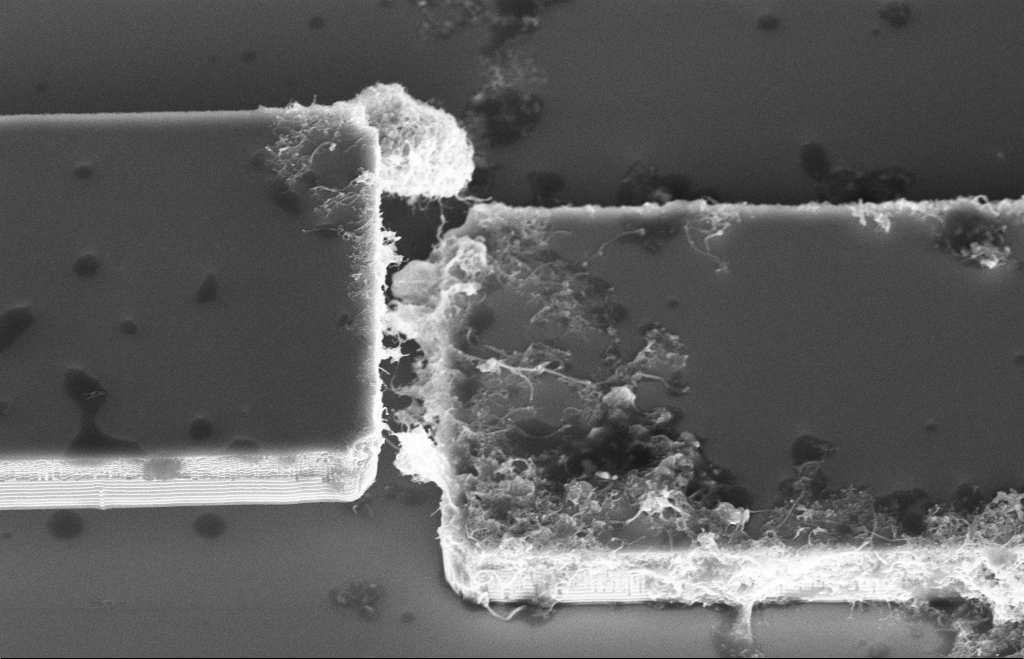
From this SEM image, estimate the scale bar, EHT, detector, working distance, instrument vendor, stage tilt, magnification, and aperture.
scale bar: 2000 nm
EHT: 10 kV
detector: InLens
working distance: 10 mm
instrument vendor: Zeiss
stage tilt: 36.3°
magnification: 8.63 K X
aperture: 20 µm